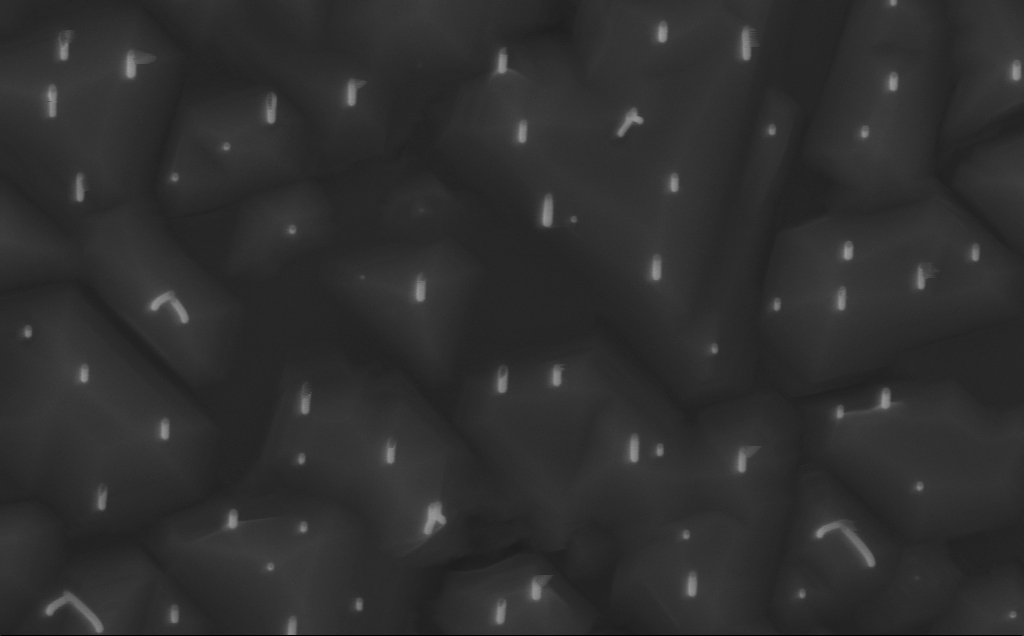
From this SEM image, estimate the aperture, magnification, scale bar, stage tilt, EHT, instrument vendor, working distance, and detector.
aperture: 30 µm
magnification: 80 K X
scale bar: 200 nm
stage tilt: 0°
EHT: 10 kV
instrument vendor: Zeiss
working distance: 4 mm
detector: InLens